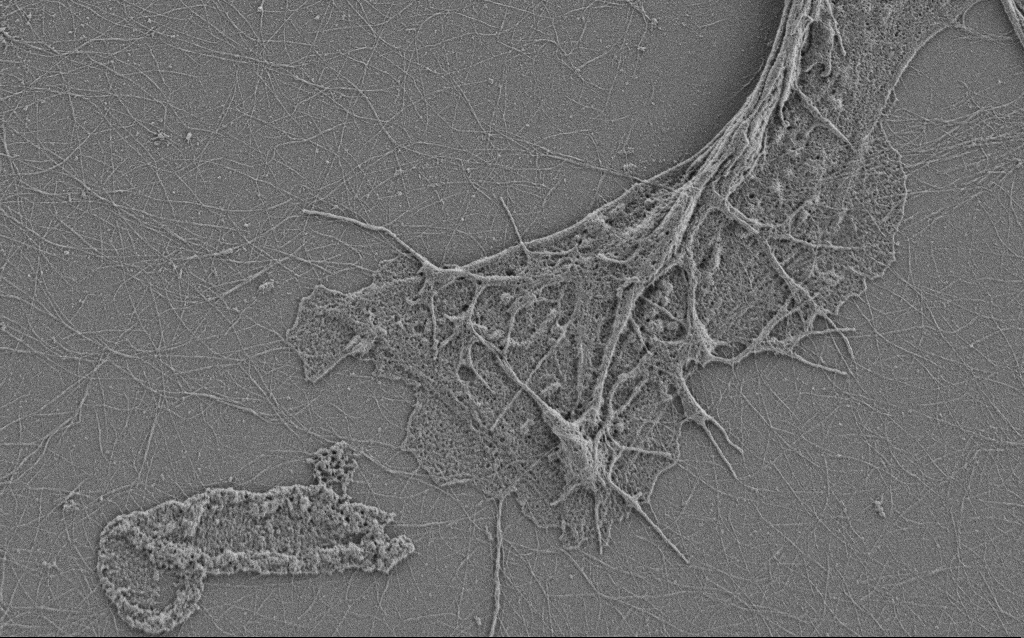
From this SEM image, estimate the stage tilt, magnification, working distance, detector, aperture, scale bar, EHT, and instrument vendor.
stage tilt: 0°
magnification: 10 K X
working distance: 4 mm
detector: SE2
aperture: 30 µm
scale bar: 2000 nm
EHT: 0.9 kV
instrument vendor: Zeiss